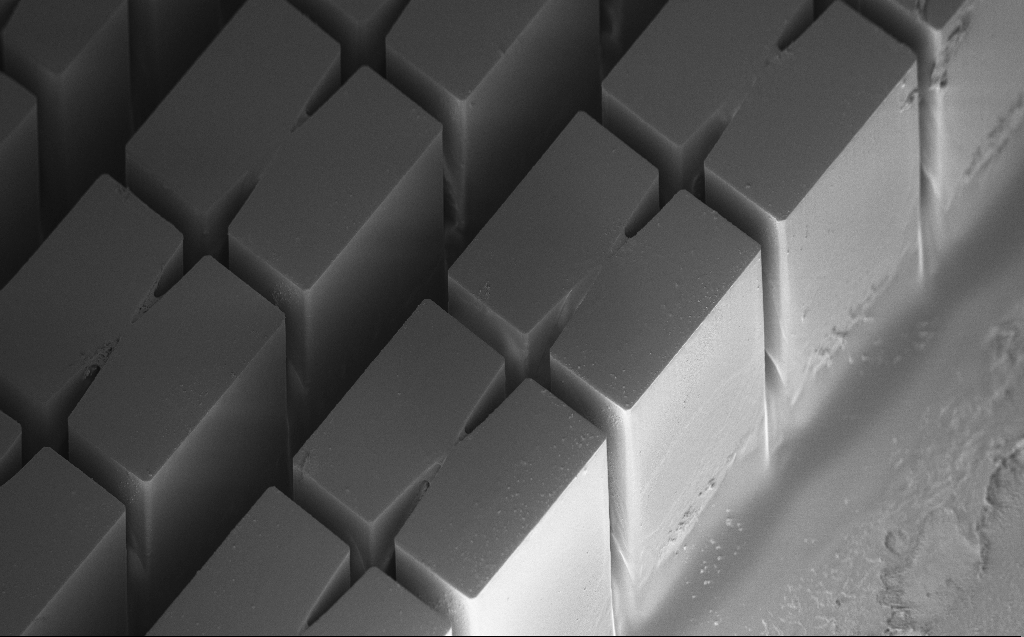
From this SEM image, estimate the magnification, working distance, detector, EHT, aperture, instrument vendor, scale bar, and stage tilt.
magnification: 0.249 K X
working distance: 7 mm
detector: InLens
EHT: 3 kV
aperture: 30 µm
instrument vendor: Zeiss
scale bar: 200000 nm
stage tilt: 45°